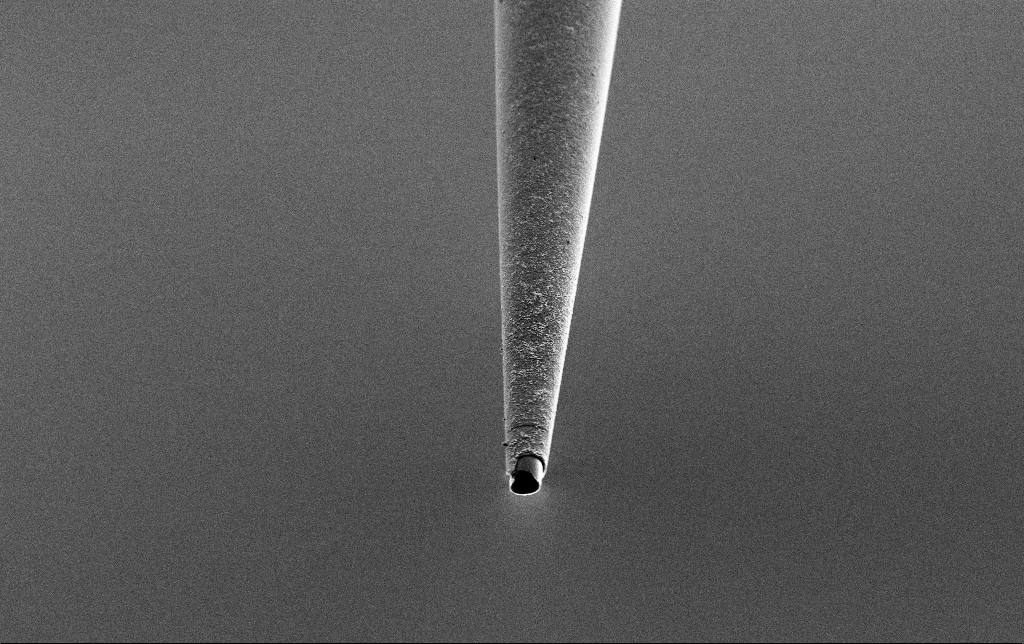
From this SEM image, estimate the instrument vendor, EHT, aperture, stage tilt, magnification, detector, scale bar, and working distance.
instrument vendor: Zeiss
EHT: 2 kV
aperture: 30 µm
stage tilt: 45°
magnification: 5 K X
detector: SE2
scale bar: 10000 nm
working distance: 7.6 mm